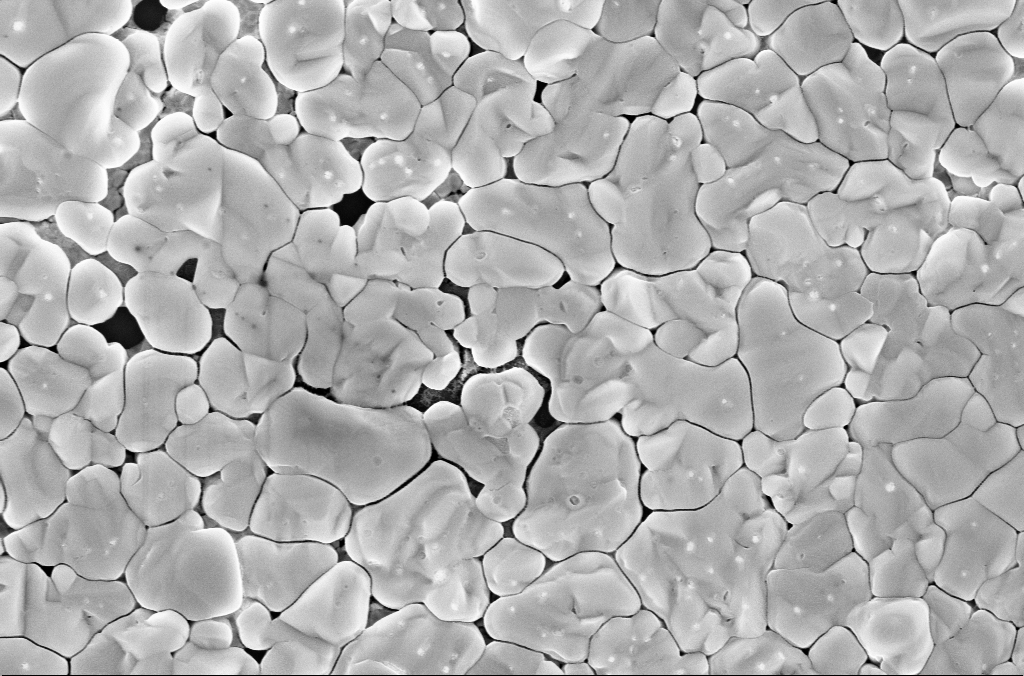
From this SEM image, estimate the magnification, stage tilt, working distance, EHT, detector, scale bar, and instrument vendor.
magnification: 40 K X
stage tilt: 0°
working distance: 3.1 mm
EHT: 5 kV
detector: InLens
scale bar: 1000 nm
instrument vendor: Zeiss